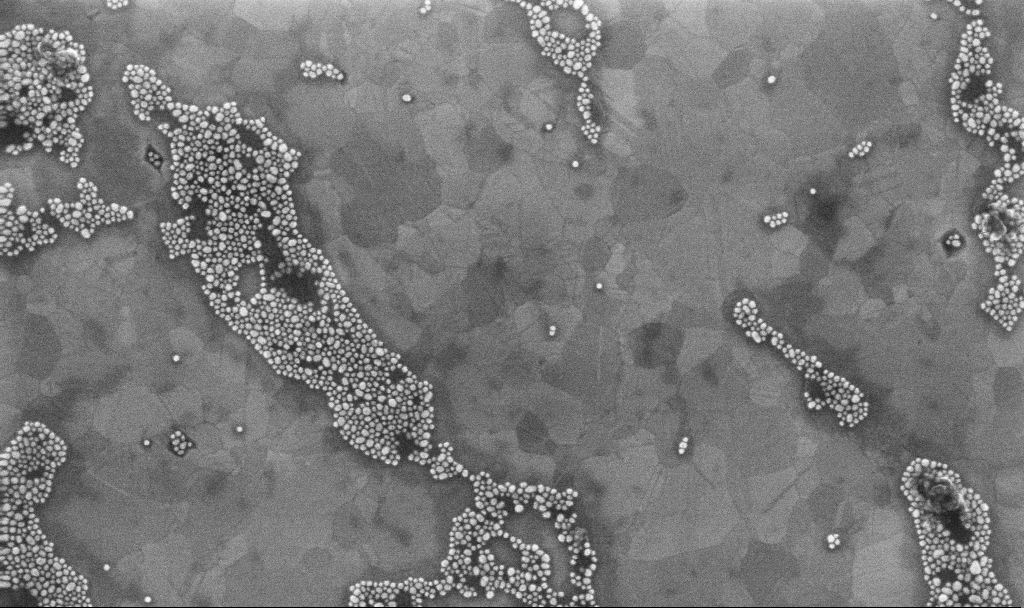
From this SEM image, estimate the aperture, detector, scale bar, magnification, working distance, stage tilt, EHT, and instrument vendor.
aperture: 30 µm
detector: InLens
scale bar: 200 nm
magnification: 110.18 K X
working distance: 3.1 mm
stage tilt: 0°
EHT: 10 kV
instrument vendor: Zeiss